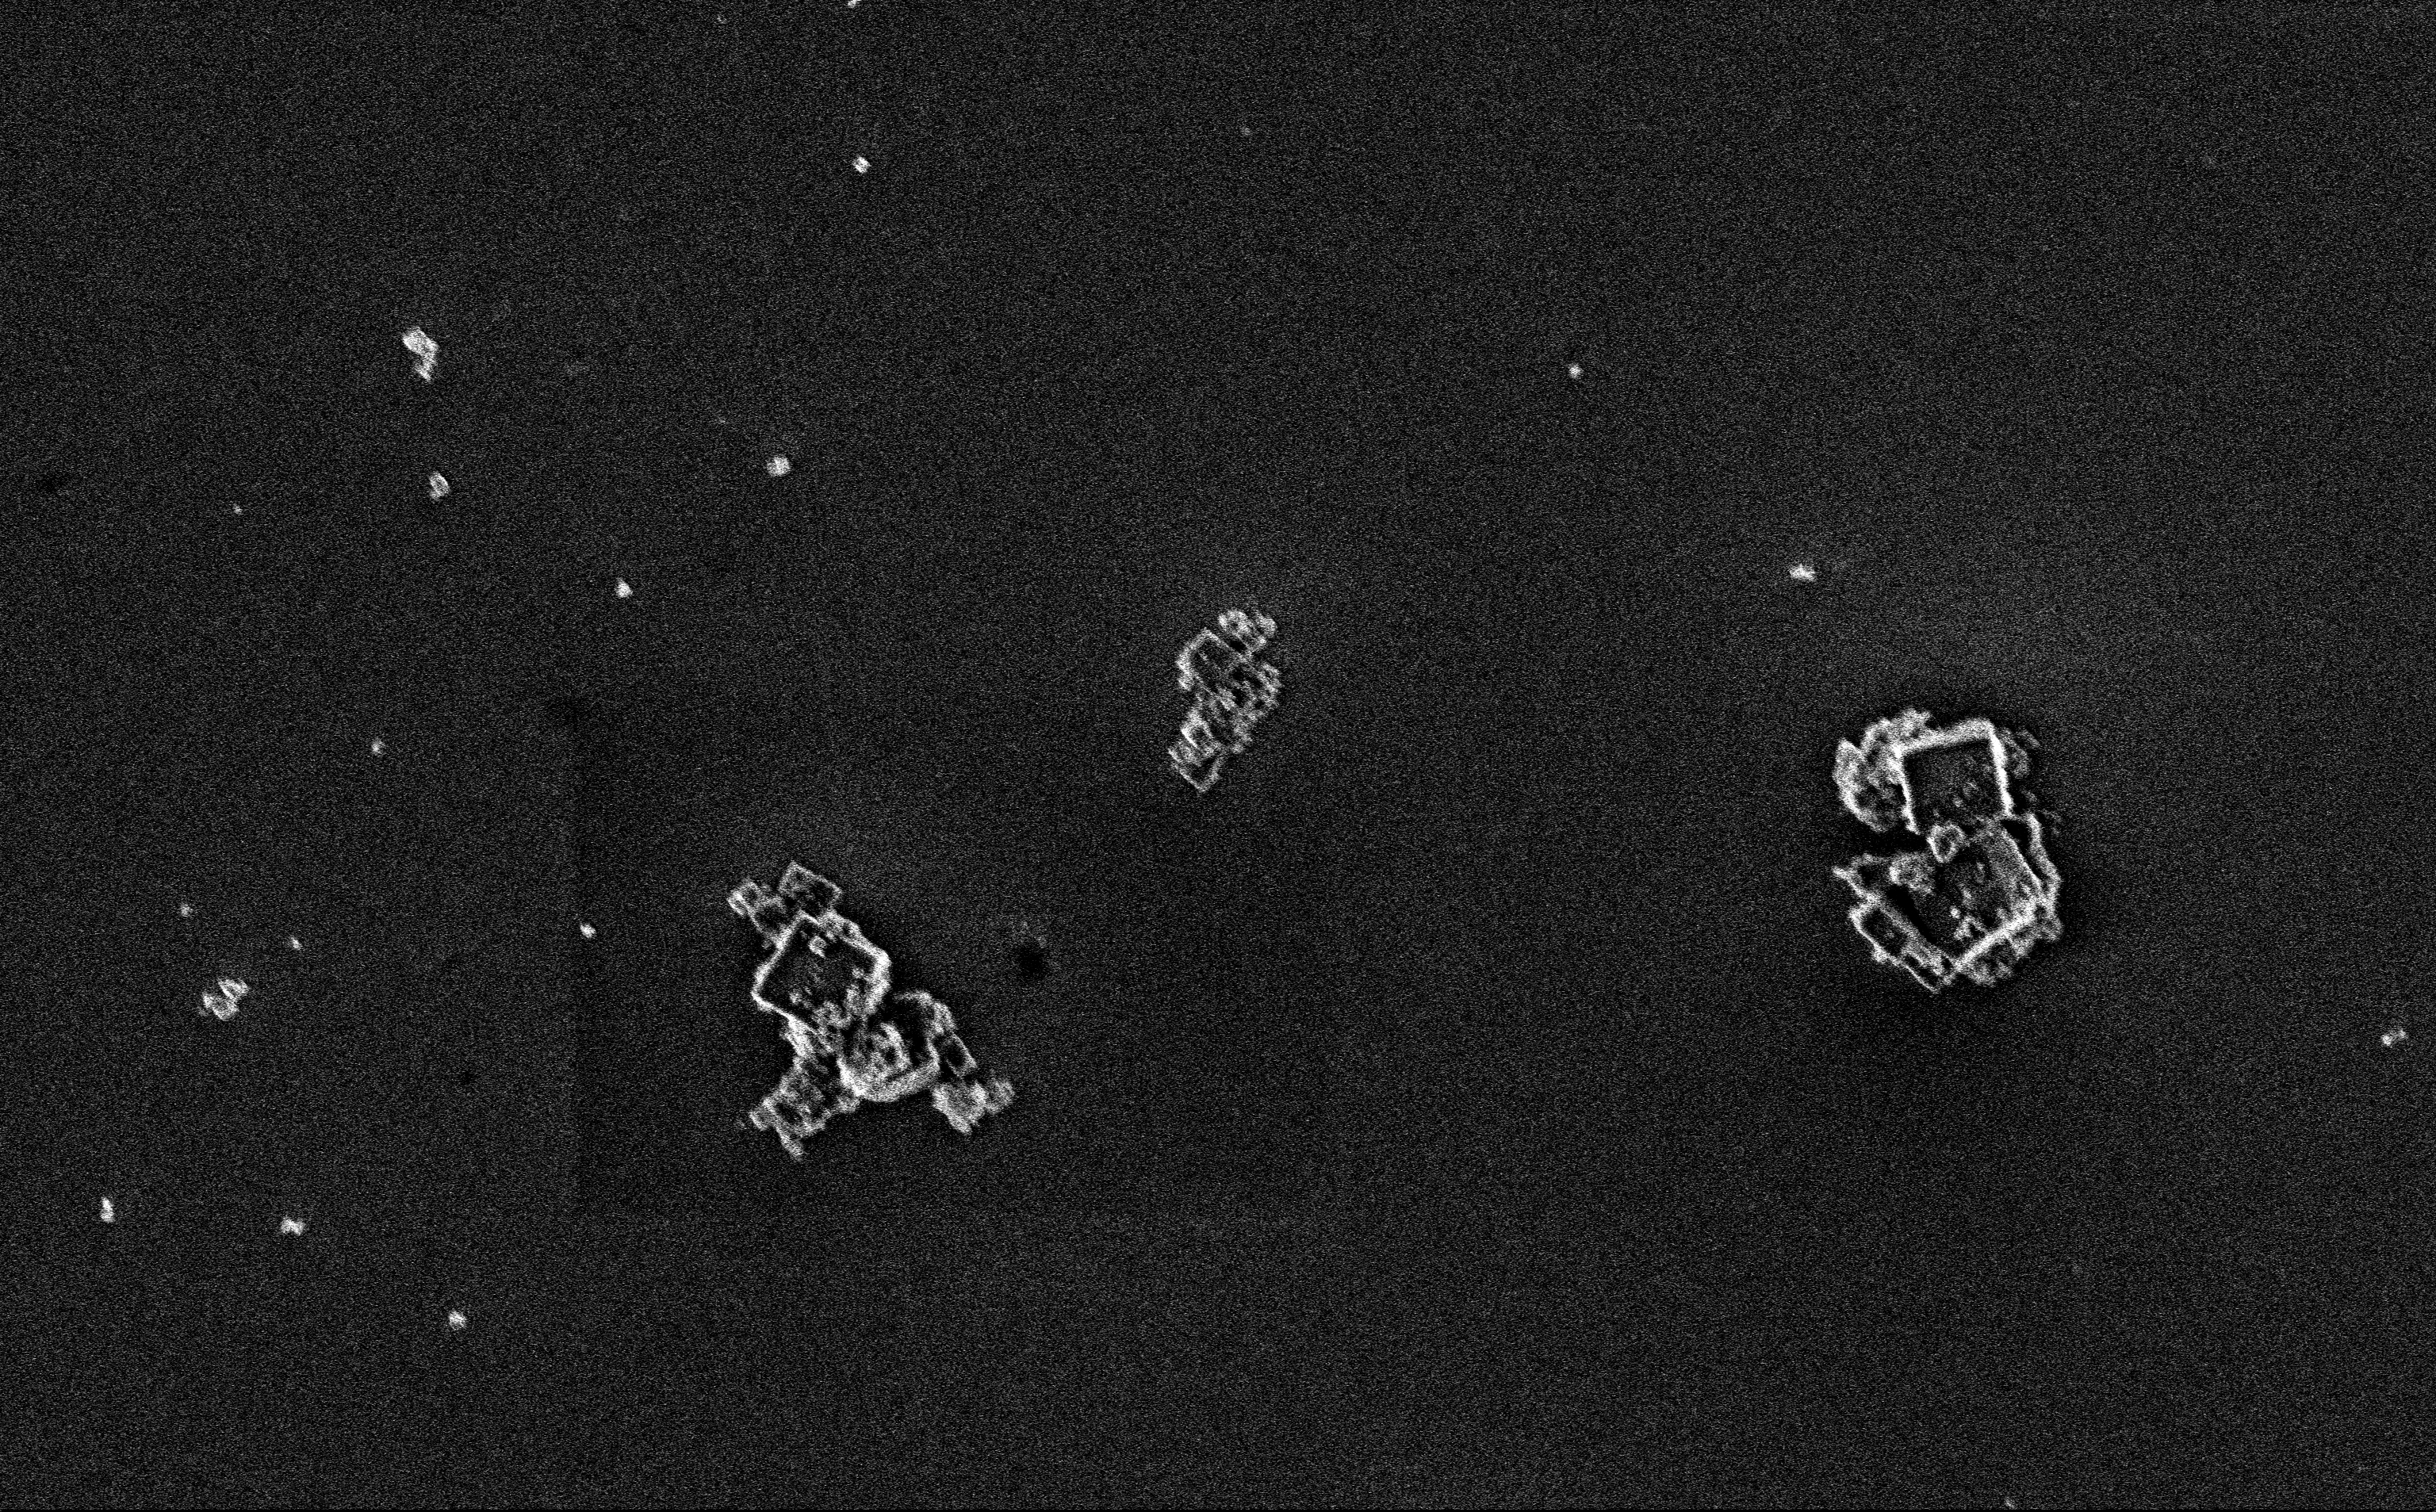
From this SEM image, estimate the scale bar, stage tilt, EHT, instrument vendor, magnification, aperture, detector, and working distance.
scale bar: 2000 nm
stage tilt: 0°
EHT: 3 kV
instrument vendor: Zeiss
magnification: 11.11 K X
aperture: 30 µm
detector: InLens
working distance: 3 mm